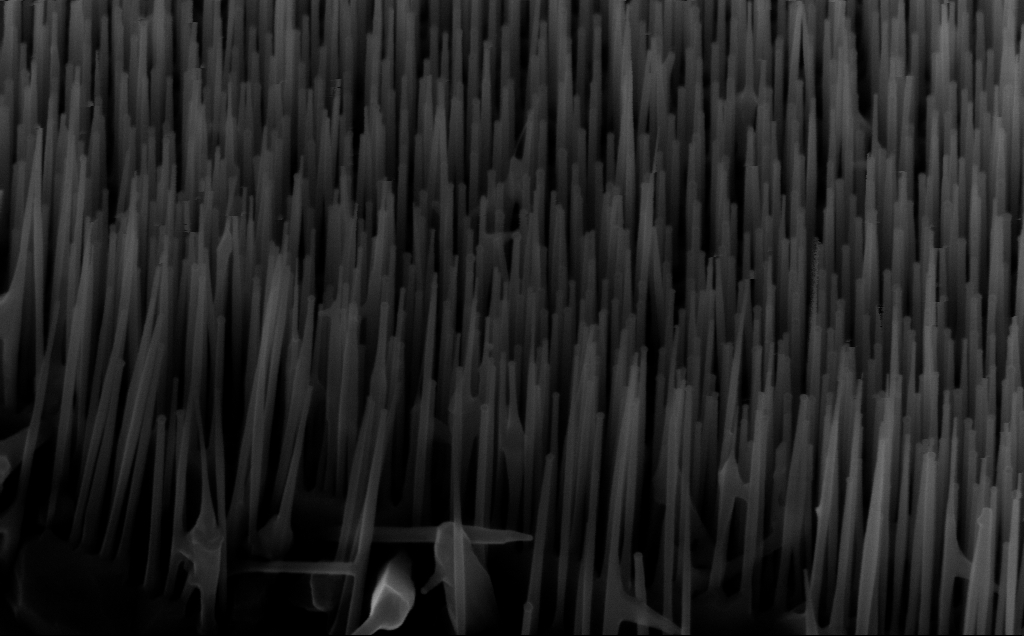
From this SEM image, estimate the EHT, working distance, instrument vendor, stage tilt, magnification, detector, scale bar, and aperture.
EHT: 10 kV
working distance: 5 mm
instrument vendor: Zeiss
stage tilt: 45°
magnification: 80 K X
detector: InLens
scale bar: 200 nm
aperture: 30 µm